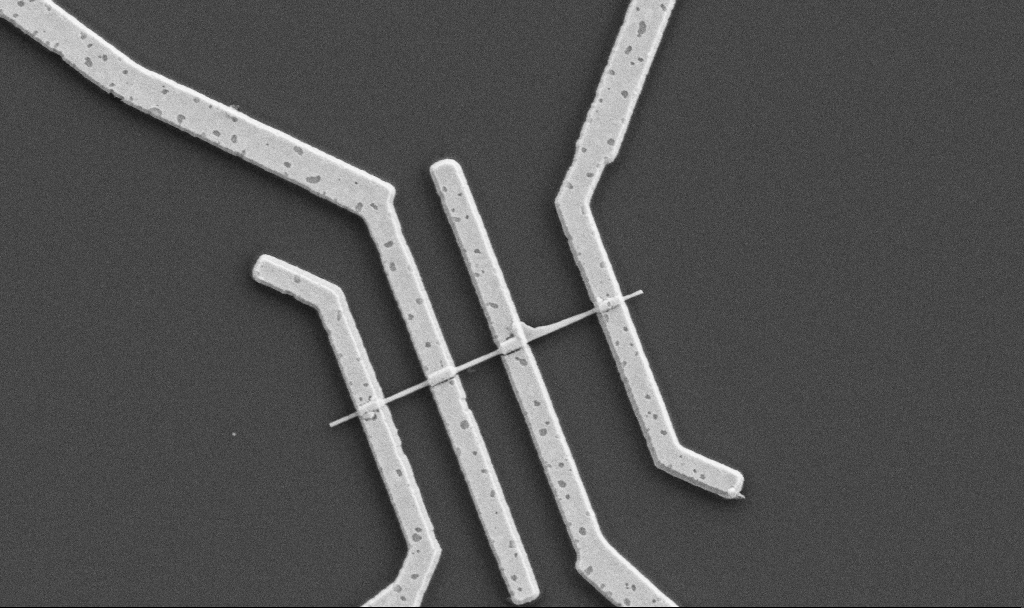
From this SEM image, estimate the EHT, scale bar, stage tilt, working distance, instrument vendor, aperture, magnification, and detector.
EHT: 5 kV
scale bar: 1000 nm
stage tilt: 0°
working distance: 10.6 mm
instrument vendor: Zeiss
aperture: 30 µm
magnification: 20 K X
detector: SE2